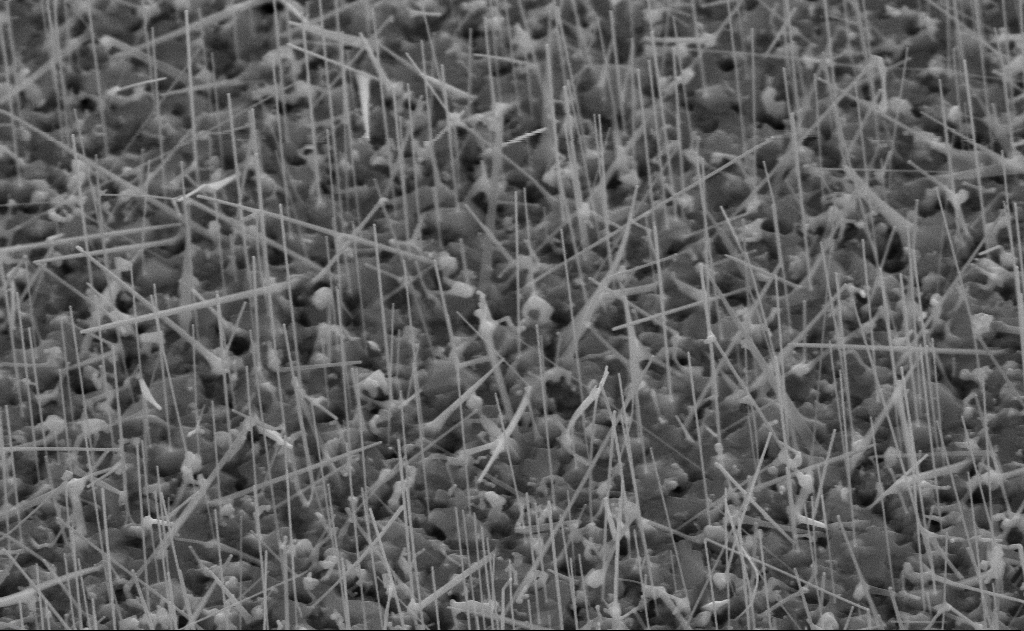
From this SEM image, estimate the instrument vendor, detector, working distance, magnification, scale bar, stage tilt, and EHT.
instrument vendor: Zeiss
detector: SE2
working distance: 11 mm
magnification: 40 K X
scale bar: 1000 nm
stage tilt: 45°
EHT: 10 kV